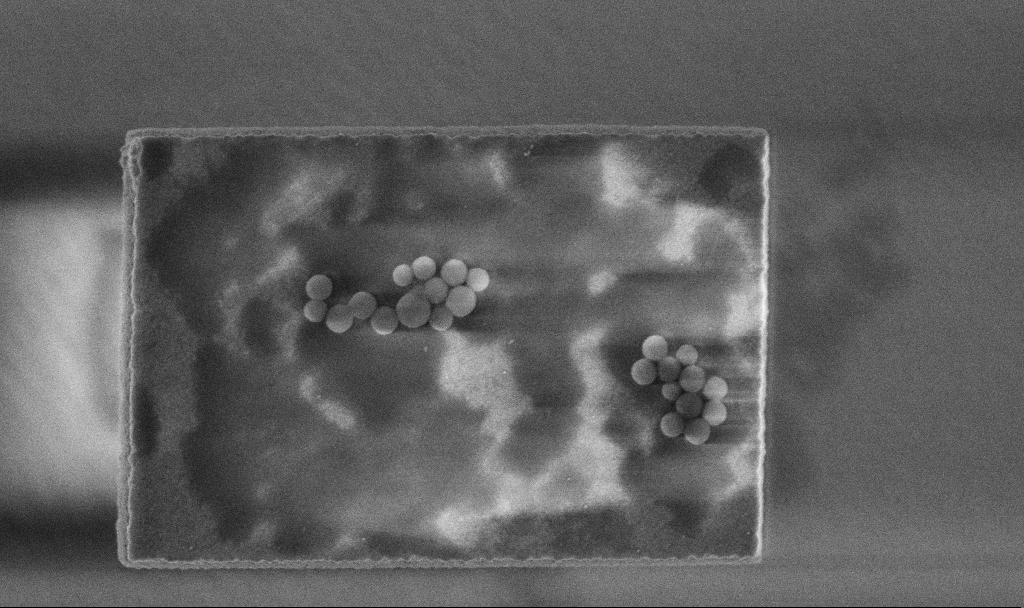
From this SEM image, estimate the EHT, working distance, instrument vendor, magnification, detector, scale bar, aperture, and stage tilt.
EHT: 3 kV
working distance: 3.3 mm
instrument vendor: Zeiss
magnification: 53.03 K X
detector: InLens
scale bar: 1000 nm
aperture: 30 µm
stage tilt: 0°